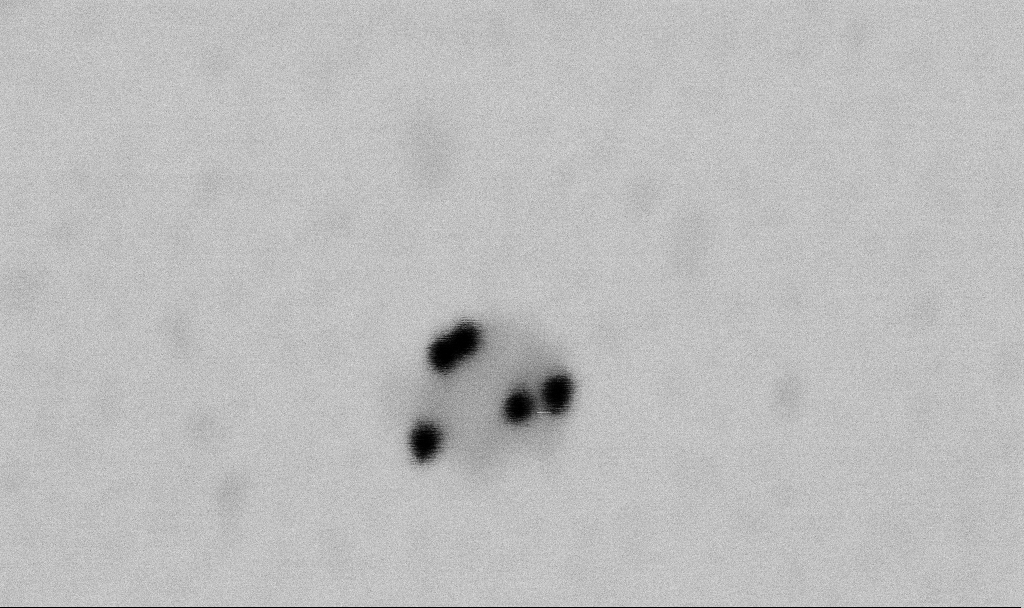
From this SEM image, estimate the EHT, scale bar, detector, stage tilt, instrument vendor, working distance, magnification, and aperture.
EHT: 2 kV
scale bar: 100 nm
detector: SE2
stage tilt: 0°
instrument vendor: Zeiss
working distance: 6.5 mm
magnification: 400 K X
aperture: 30 µm